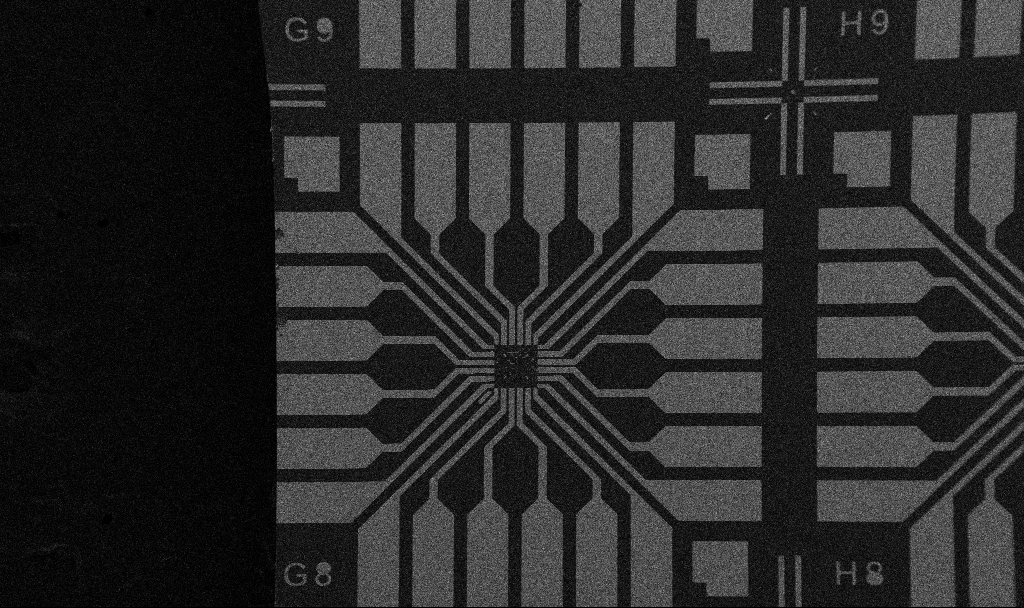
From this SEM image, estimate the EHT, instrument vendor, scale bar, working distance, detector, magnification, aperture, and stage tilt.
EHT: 5 kV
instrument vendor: Zeiss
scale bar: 200000 nm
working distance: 10.7 mm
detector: SE2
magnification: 0.1 K X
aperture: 30 µm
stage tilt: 0°